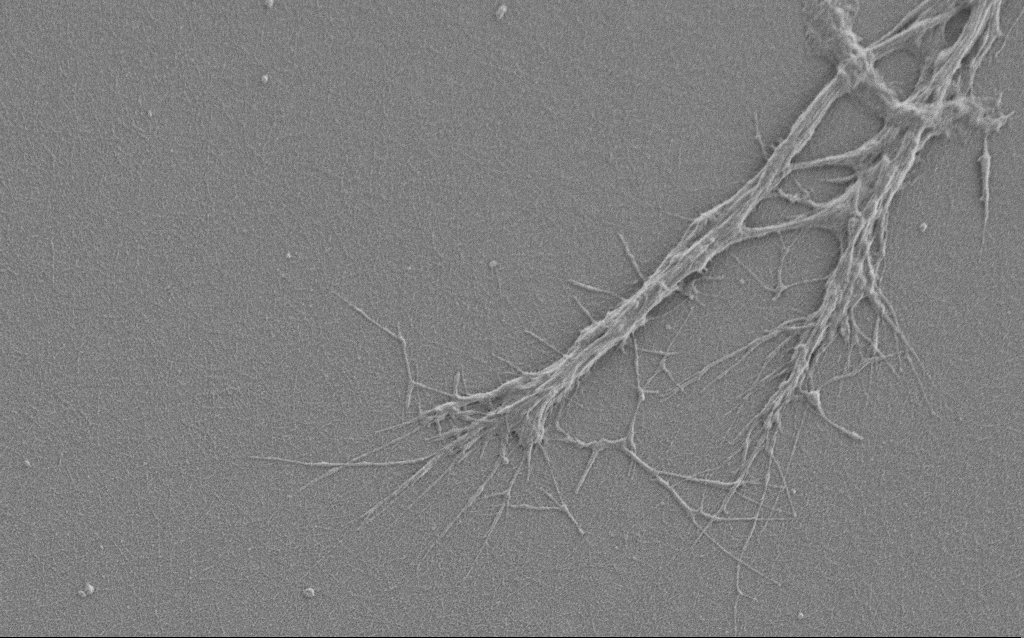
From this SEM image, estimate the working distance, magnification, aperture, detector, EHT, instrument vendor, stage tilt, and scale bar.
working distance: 6 mm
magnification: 6 K X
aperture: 30 µm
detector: SE2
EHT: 1 kV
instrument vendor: Zeiss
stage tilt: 0°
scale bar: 10000 nm